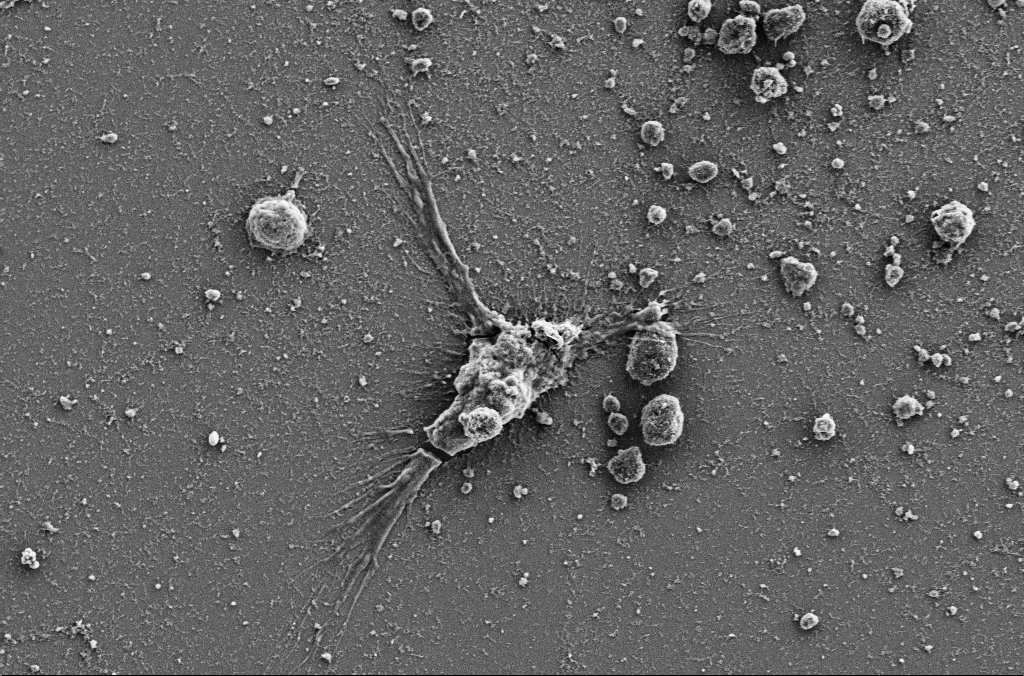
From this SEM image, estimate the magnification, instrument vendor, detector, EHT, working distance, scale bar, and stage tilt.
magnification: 3 K X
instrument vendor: Zeiss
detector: SE2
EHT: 5 kV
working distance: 3.9 mm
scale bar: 10000 nm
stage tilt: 0°